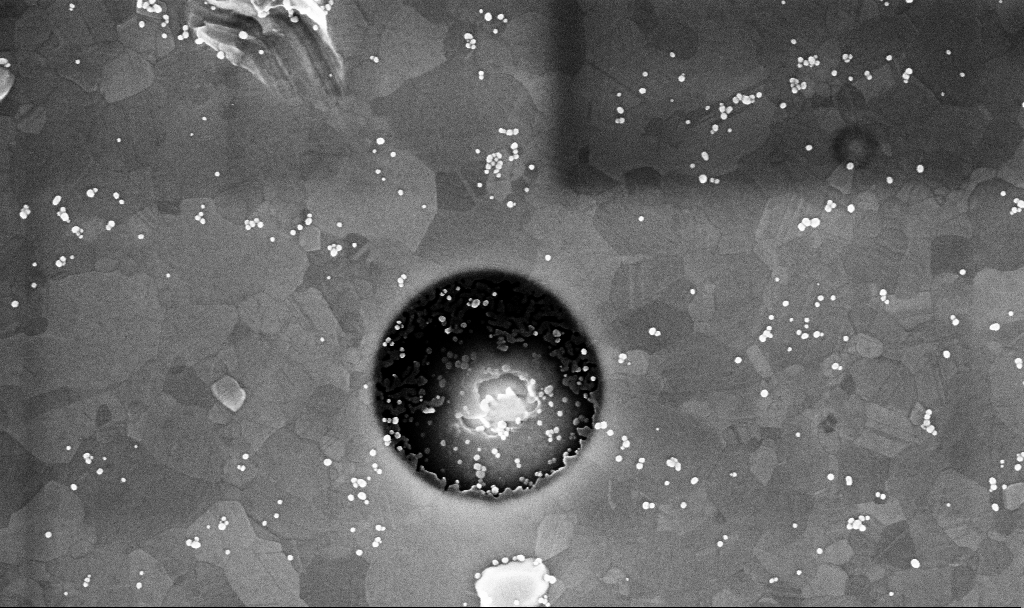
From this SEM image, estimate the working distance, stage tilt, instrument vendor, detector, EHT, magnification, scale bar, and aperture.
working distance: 3.4 mm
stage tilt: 0°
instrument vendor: Zeiss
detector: InLens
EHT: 10 kV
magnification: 100.42 K X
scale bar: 200 nm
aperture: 30 µm